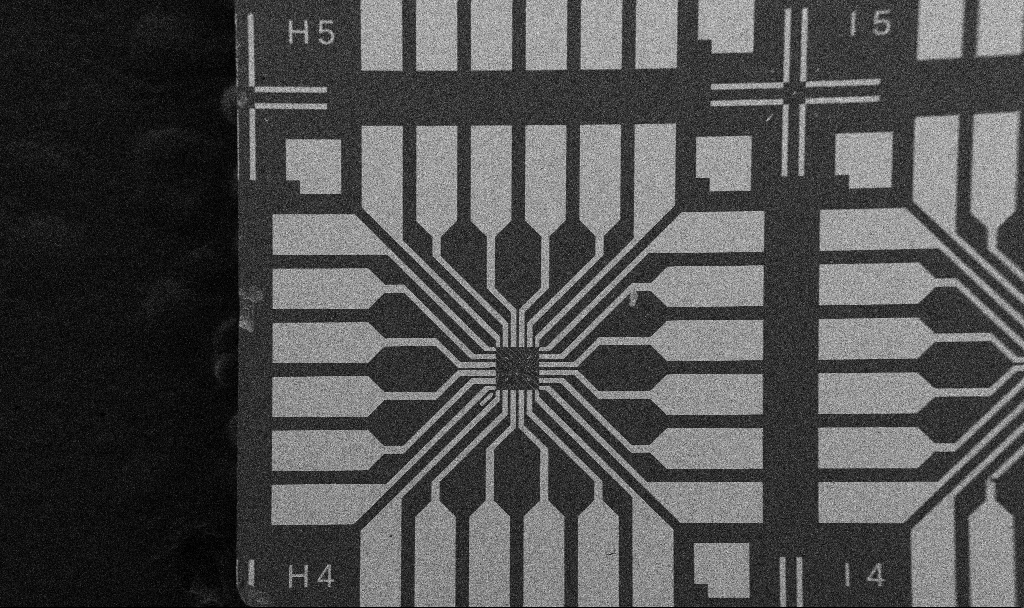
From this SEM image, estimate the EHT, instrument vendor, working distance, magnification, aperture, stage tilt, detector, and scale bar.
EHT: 5 kV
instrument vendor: Zeiss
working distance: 10.7 mm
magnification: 0.1 K X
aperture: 30 µm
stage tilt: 0°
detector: SE2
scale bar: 200000 nm